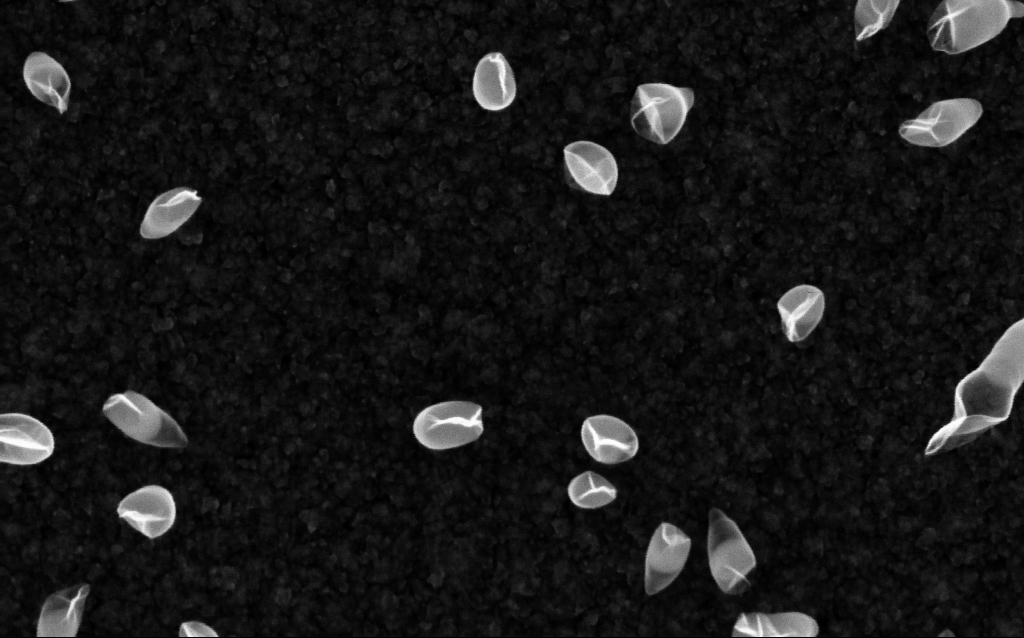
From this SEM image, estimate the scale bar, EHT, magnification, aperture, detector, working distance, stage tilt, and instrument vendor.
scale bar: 100 nm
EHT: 5 kV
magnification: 200 K X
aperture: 30 µm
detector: InLens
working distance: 2.1 mm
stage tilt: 0°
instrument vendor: Zeiss